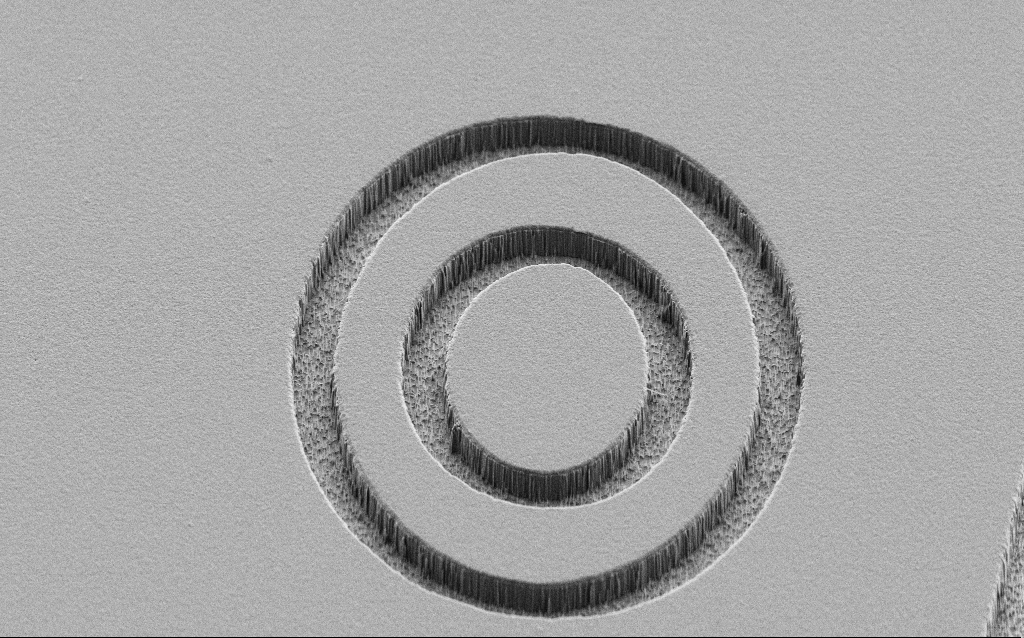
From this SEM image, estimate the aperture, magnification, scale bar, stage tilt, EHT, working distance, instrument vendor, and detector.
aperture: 30 µm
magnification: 2.03 K X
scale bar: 20000 nm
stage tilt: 45°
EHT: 3 kV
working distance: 7 mm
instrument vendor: Zeiss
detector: SE2